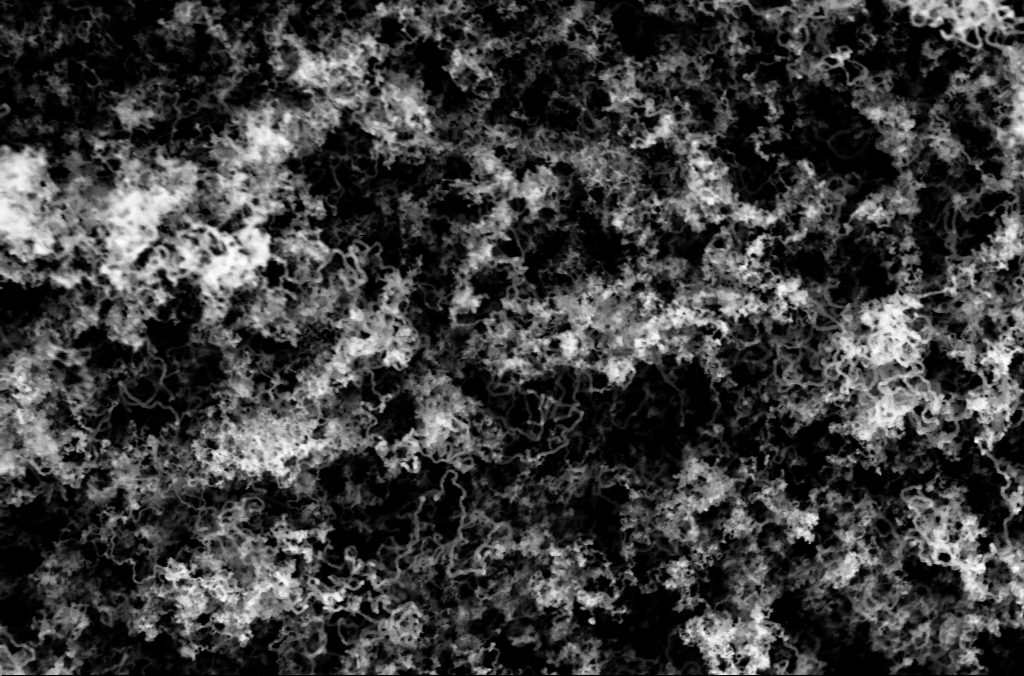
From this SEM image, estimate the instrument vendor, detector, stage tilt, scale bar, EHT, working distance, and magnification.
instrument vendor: Zeiss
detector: InLens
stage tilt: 0°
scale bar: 1000 nm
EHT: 3 kV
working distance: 5.1 mm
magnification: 50 K X